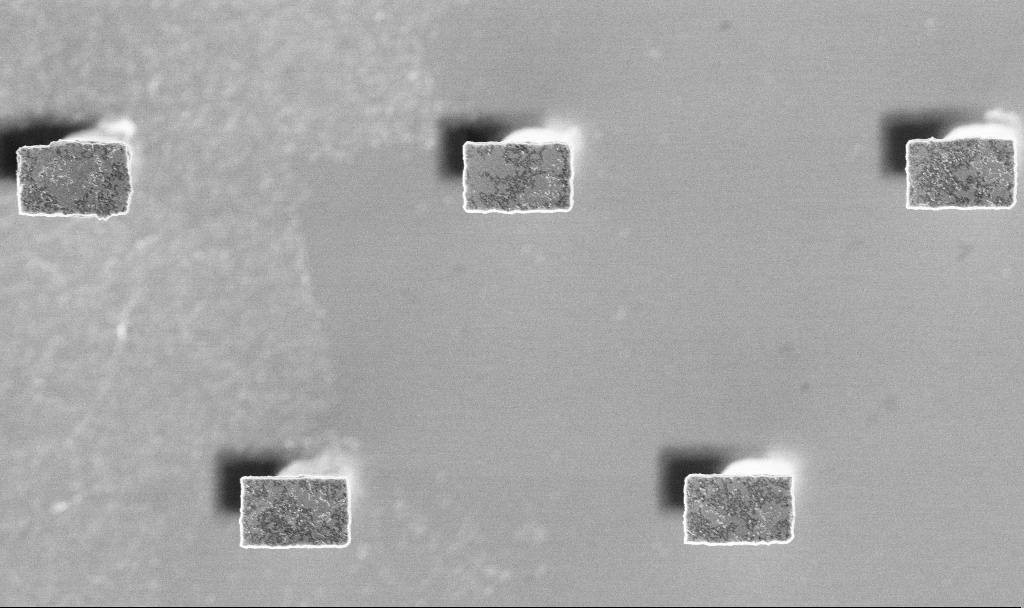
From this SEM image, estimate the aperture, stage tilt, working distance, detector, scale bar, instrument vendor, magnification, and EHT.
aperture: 30 µm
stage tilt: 0°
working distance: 3.2 mm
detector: InLens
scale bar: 2000 nm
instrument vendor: Zeiss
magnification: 13.81 K X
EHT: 3 kV